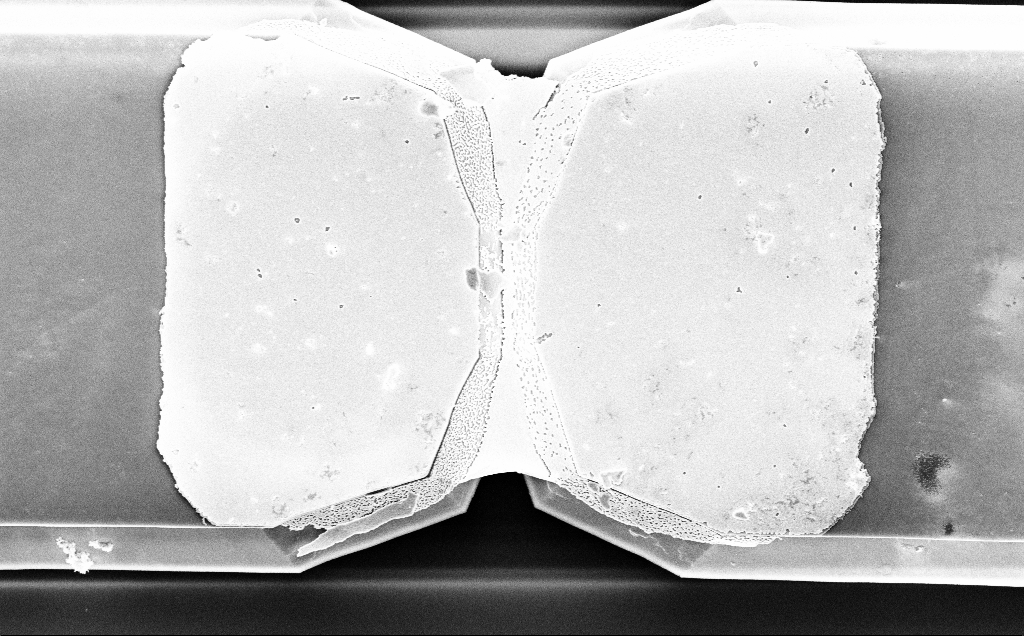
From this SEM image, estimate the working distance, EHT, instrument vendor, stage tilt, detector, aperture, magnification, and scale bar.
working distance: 15 mm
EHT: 5 kV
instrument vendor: Zeiss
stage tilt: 0.2°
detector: InLens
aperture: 30 µm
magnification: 9.81 K X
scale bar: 2000 nm